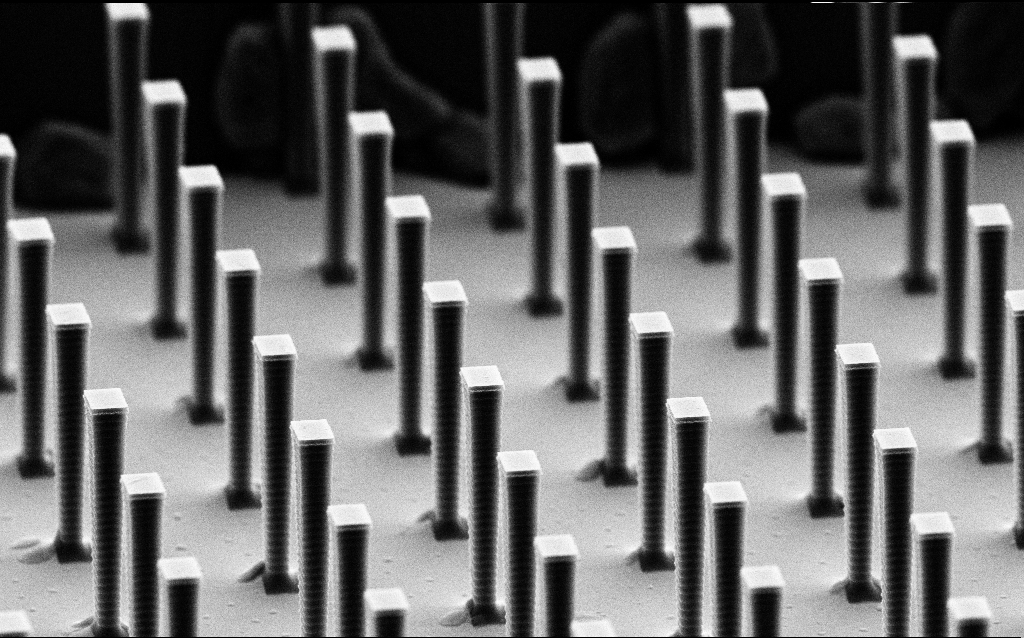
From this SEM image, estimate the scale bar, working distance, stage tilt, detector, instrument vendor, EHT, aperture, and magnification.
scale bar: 2000 nm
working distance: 6.5 mm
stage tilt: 70°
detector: SE2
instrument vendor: Zeiss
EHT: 8 kV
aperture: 30 µm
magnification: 7.74 K X